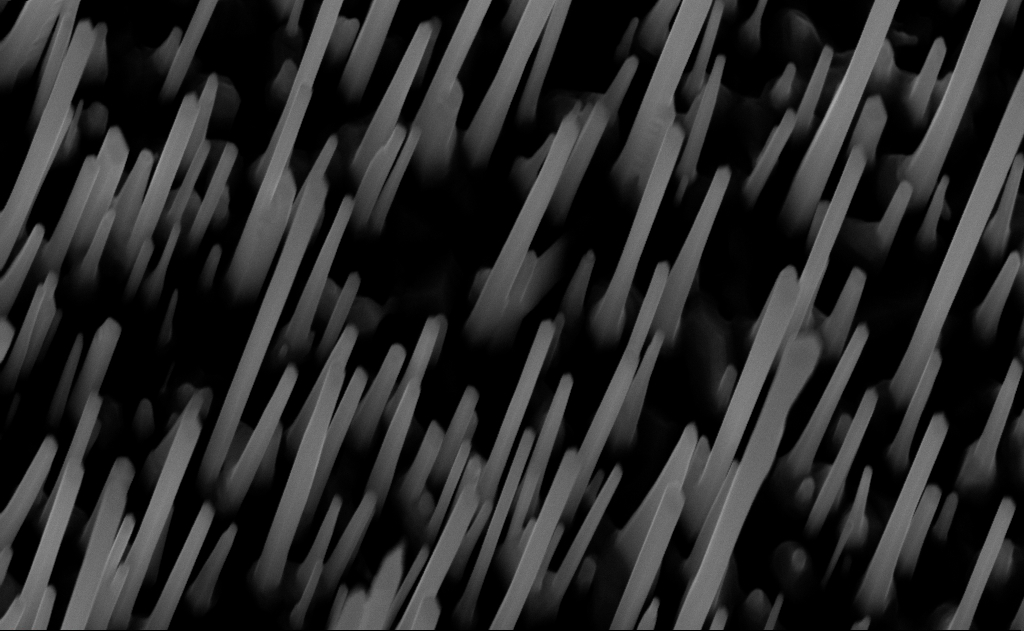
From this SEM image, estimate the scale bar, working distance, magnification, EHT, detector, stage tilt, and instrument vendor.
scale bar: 200 nm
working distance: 7 mm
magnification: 80 K X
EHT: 10 kV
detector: InLens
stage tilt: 0°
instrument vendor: Zeiss